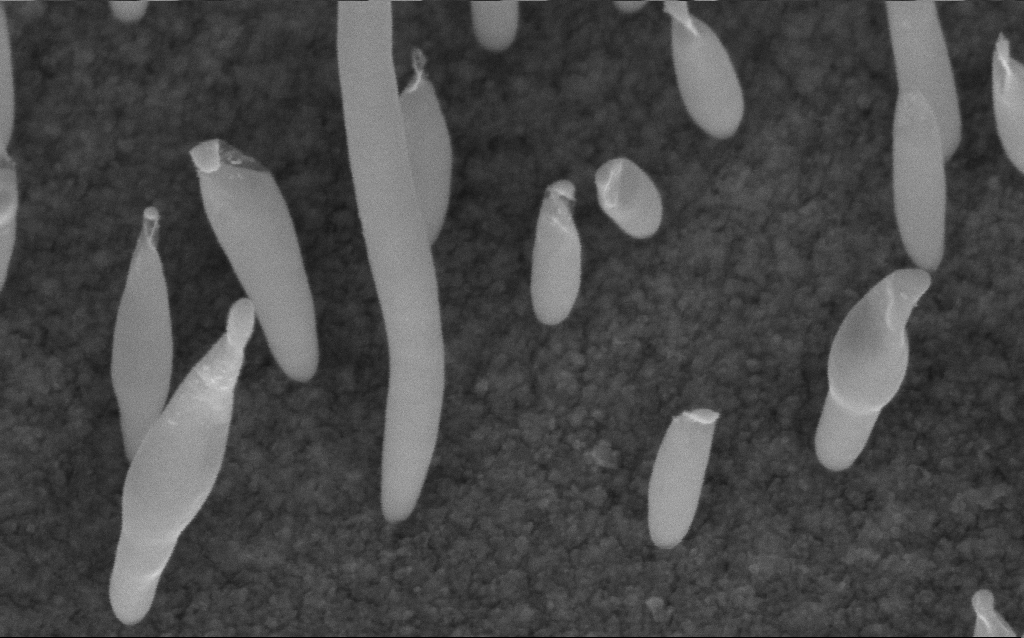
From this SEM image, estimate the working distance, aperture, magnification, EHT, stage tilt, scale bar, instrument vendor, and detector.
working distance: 7.1 mm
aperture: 30 µm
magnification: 200 K X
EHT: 5 kV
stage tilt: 45°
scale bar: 100 nm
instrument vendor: Zeiss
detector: InLens